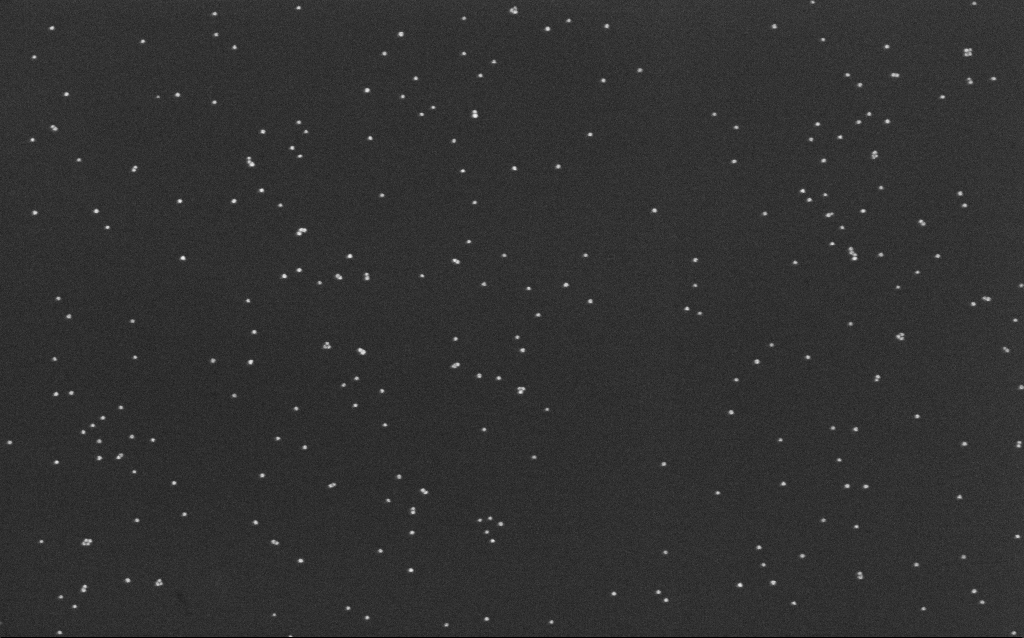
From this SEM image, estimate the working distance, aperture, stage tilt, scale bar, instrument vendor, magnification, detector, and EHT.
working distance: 6.6 mm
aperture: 30 µm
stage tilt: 0°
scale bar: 200 nm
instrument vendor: Zeiss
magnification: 100 K X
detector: InLens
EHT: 10 kV